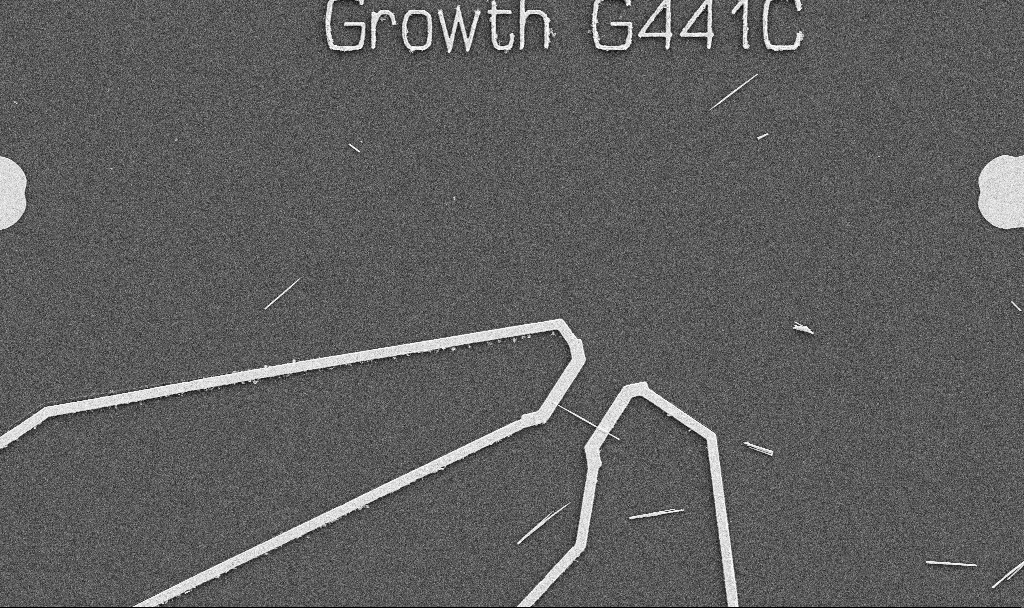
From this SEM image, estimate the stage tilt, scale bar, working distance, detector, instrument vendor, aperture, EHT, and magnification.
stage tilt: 0°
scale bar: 10000 nm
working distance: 10.7 mm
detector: SE2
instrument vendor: Zeiss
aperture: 30 µm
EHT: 5 kV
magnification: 5 K X